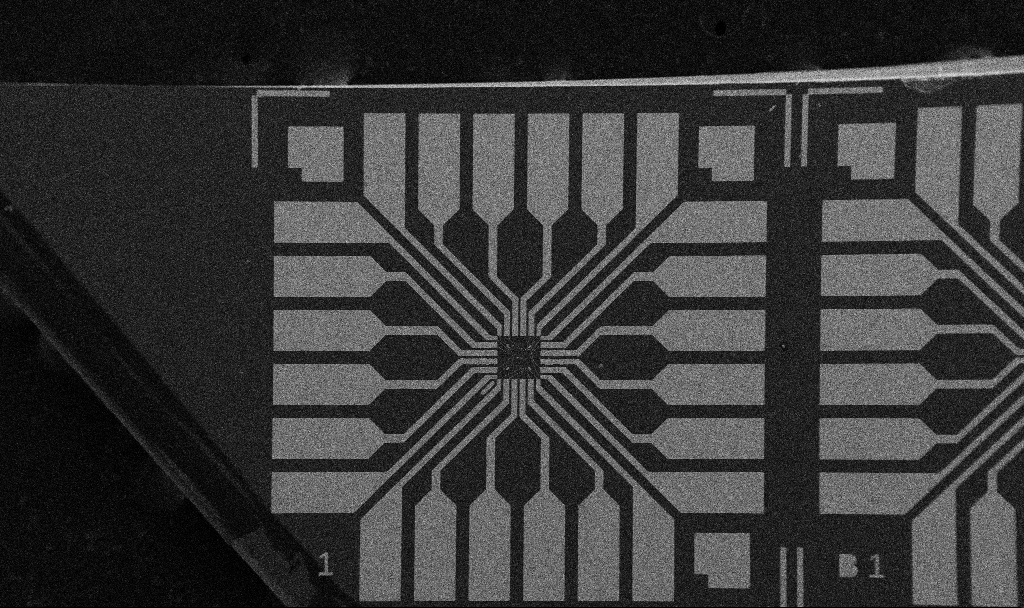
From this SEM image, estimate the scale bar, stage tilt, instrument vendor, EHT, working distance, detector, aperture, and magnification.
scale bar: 200000 nm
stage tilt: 0°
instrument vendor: Zeiss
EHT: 5 kV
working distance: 10.7 mm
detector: SE2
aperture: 30 µm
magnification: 0.1 K X